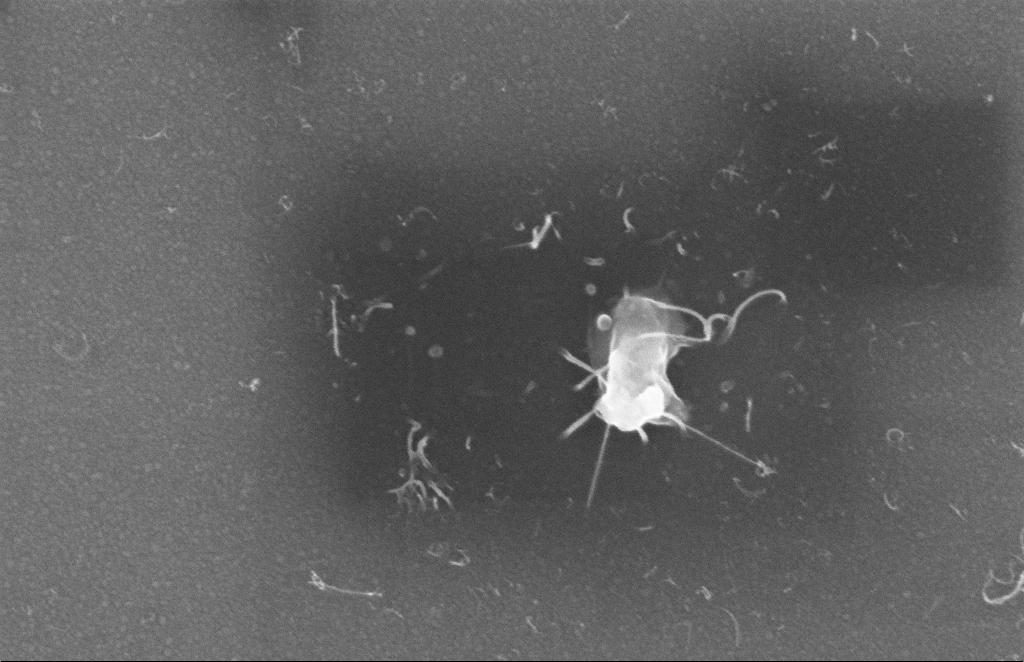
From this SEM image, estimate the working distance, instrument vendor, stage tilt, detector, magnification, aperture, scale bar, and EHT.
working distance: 8 mm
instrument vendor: Zeiss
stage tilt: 0°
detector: InLens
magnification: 126.43 K X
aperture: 20 µm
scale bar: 100 nm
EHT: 5 kV